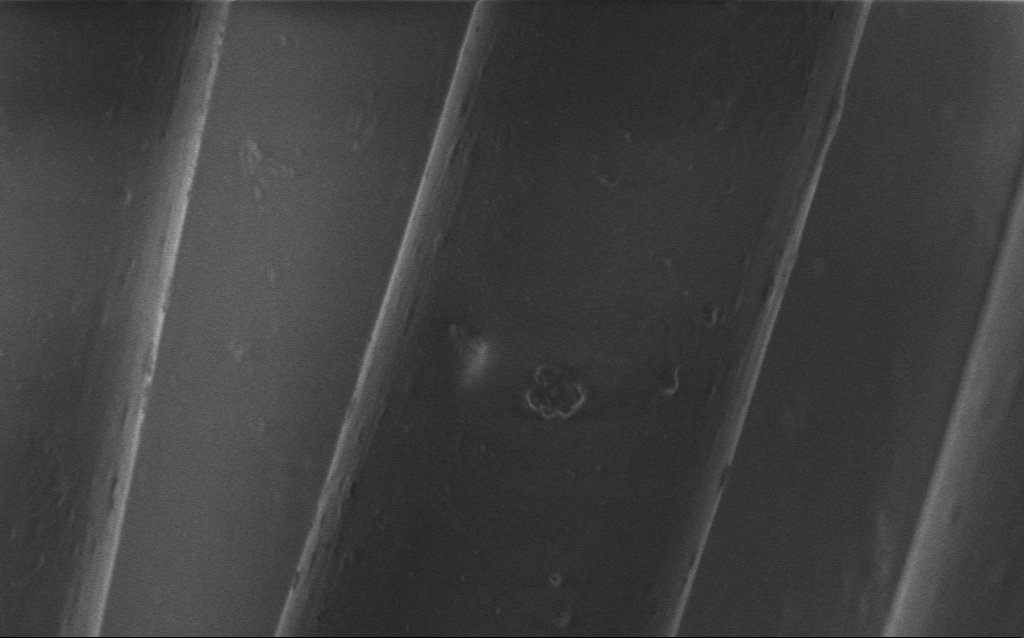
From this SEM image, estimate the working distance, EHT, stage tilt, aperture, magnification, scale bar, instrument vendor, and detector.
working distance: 4 mm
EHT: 1 kV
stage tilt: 0°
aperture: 30 µm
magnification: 5.98 K X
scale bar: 10000 nm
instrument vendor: Zeiss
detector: InLens